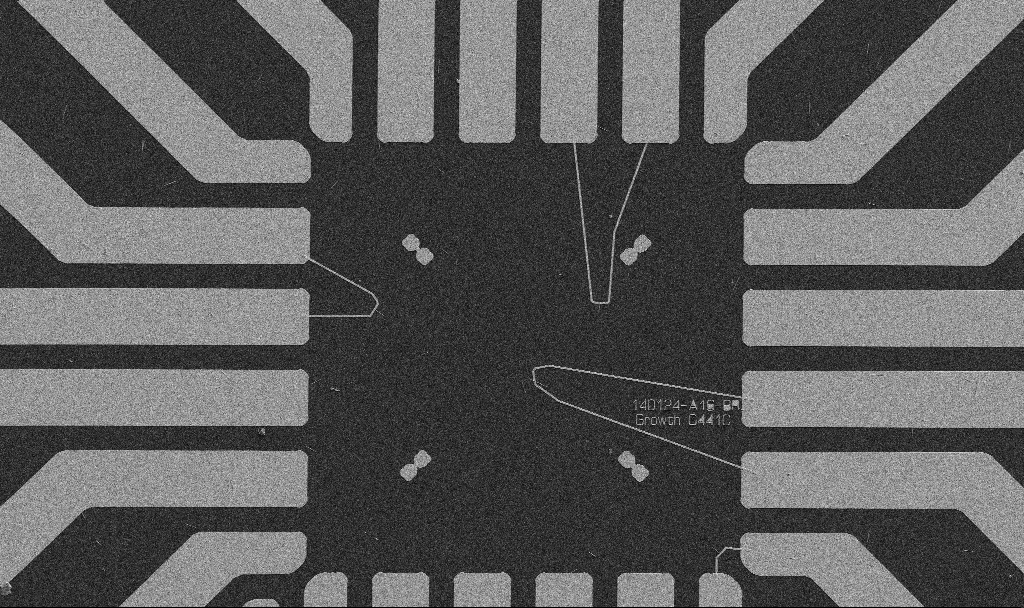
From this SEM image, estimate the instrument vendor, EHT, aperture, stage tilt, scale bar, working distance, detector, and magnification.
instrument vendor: Zeiss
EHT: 5 kV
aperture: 30 µm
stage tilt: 0°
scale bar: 20000 nm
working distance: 10.7 mm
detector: SE2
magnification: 1 K X